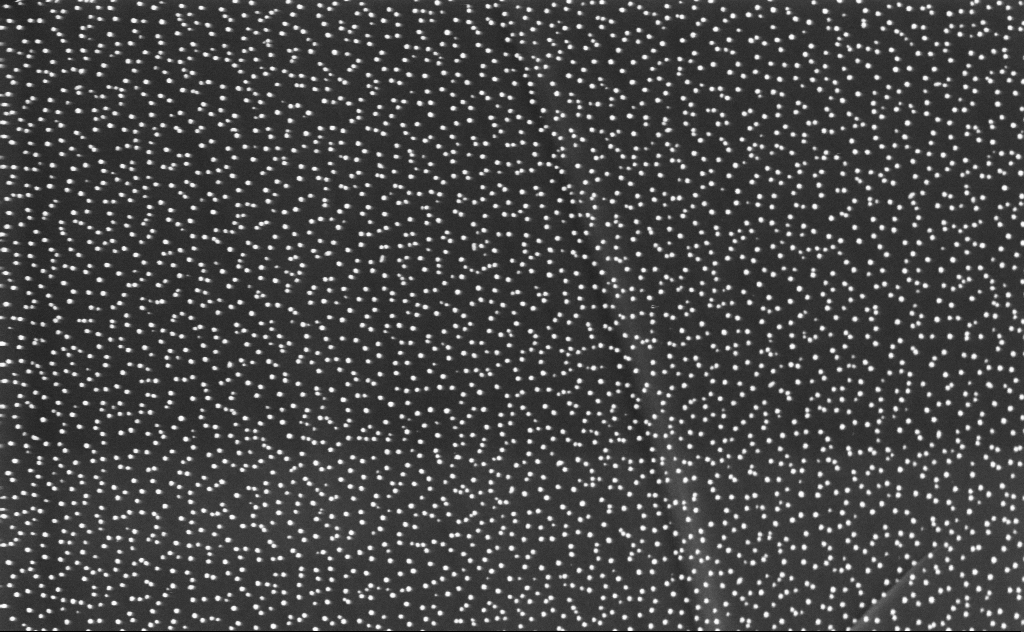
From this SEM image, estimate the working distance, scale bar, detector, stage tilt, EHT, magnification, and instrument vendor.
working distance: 5 mm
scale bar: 200 nm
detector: InLens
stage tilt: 0°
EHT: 3 kV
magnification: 80 K X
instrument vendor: Zeiss